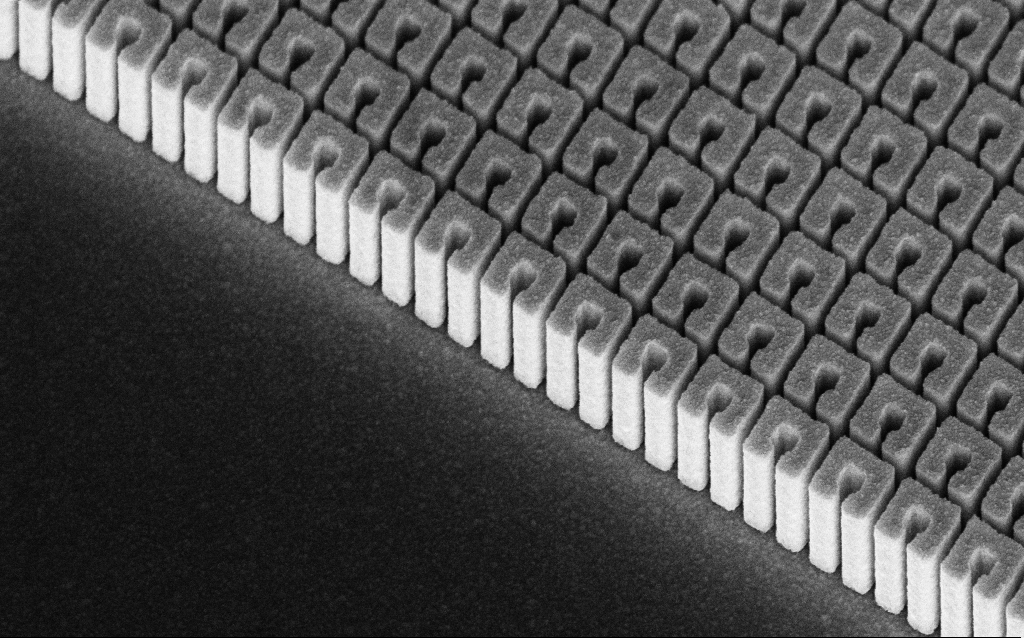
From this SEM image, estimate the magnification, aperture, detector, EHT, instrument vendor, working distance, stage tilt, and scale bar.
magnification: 61.56 K X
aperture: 30 µm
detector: InLens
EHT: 8 kV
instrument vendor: Zeiss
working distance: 10 mm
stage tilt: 45°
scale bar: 1000 nm